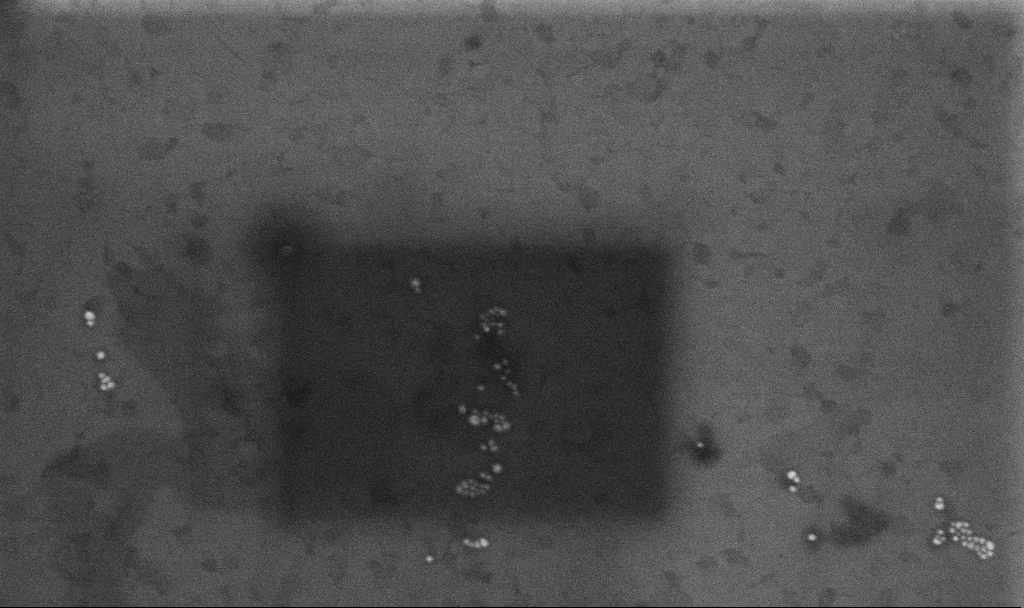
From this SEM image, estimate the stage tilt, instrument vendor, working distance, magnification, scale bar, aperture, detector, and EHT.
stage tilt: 0°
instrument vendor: Zeiss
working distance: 3.3 mm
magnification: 108.38 K X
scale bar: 200 nm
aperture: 30 µm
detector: InLens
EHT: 10 kV